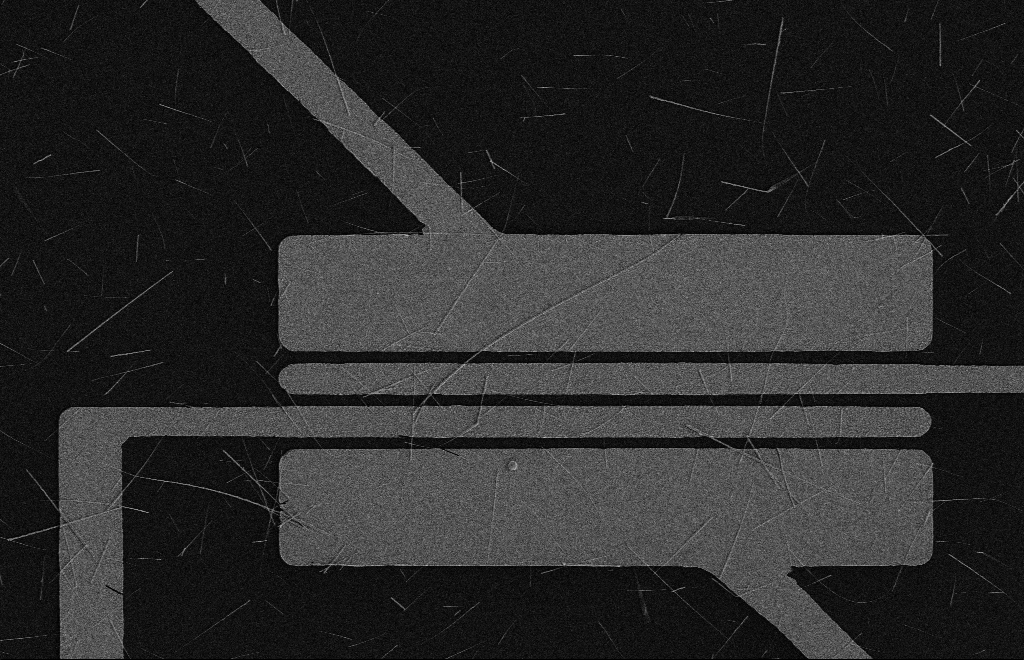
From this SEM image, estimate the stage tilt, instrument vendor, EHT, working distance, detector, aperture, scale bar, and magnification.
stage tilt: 0°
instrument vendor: Zeiss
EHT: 5 kV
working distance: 16 mm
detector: SE2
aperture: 10 µm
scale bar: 10000 nm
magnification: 3.96 K X